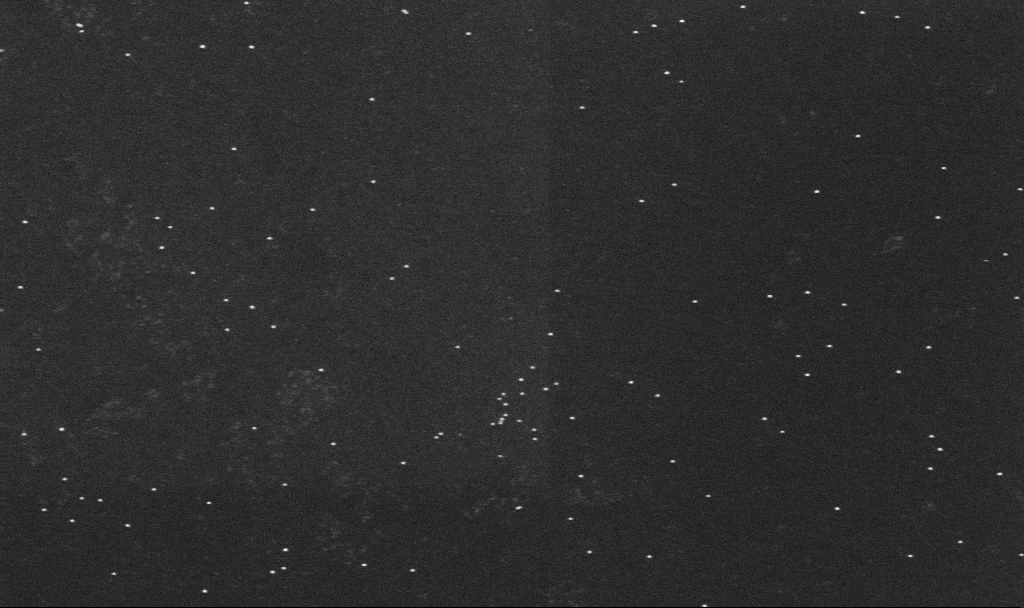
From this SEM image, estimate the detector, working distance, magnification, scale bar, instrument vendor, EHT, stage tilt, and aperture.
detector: InLens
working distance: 3.2 mm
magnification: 100 K X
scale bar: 200 nm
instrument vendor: Zeiss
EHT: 10 kV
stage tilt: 0°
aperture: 30 µm